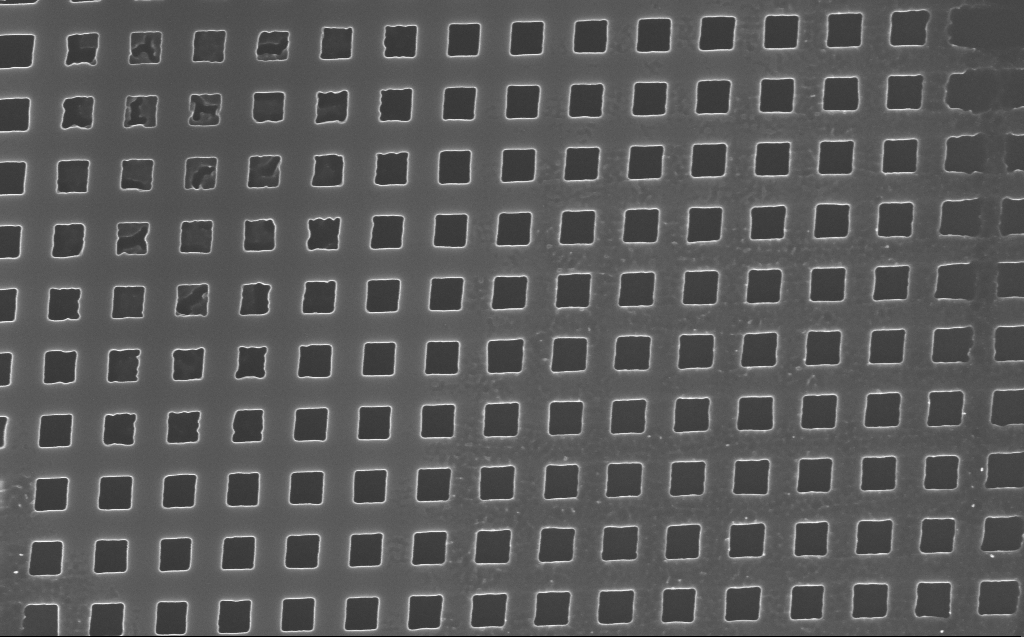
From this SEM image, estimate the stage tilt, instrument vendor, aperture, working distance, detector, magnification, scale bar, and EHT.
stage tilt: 0°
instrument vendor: Zeiss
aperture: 30 µm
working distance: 4 mm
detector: InLens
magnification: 47.06 K X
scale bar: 1000 nm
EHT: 10 kV